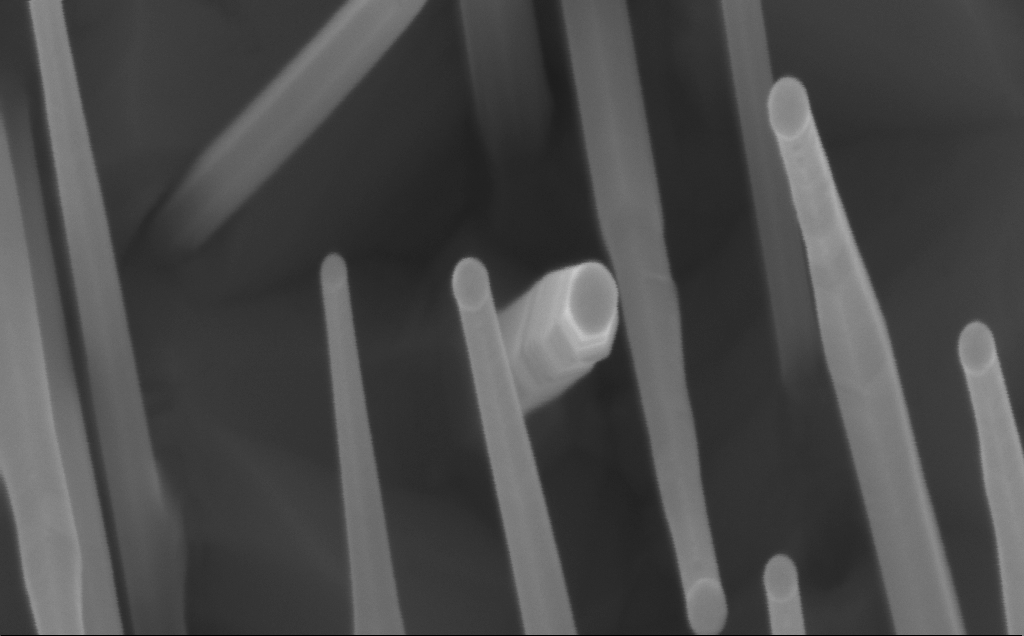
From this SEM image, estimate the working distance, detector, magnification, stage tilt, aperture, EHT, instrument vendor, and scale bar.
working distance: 7 mm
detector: InLens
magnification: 285.42 K X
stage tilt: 0°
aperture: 30 µm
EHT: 10 kV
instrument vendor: Zeiss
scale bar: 200 nm